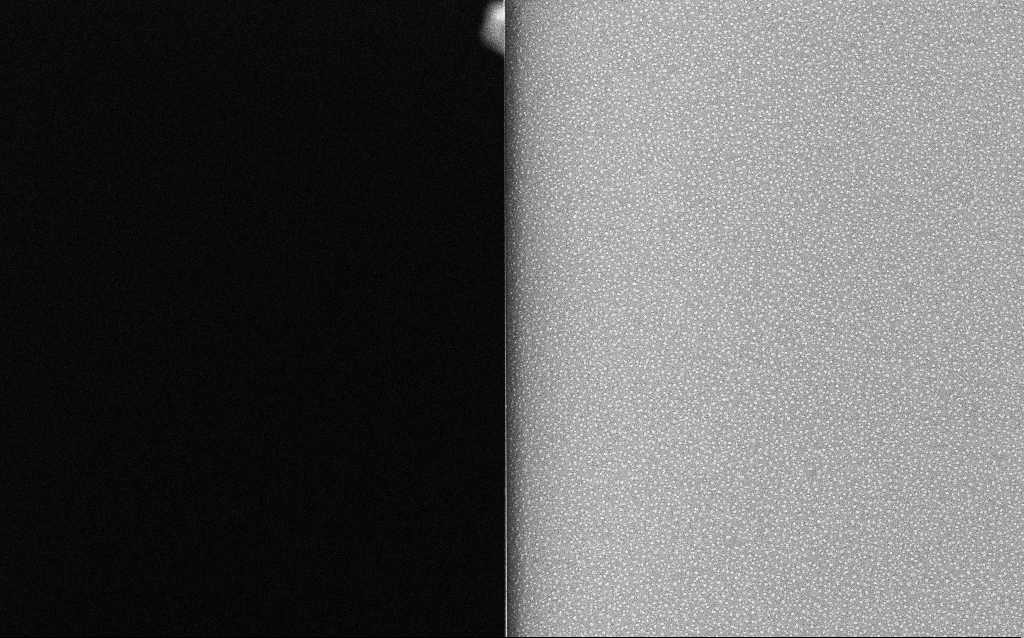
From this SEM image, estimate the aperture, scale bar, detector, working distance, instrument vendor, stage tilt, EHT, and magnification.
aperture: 30 µm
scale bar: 20000 nm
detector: InLens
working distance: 1.5 mm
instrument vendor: Zeiss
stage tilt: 0°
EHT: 20 kV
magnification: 1 K X